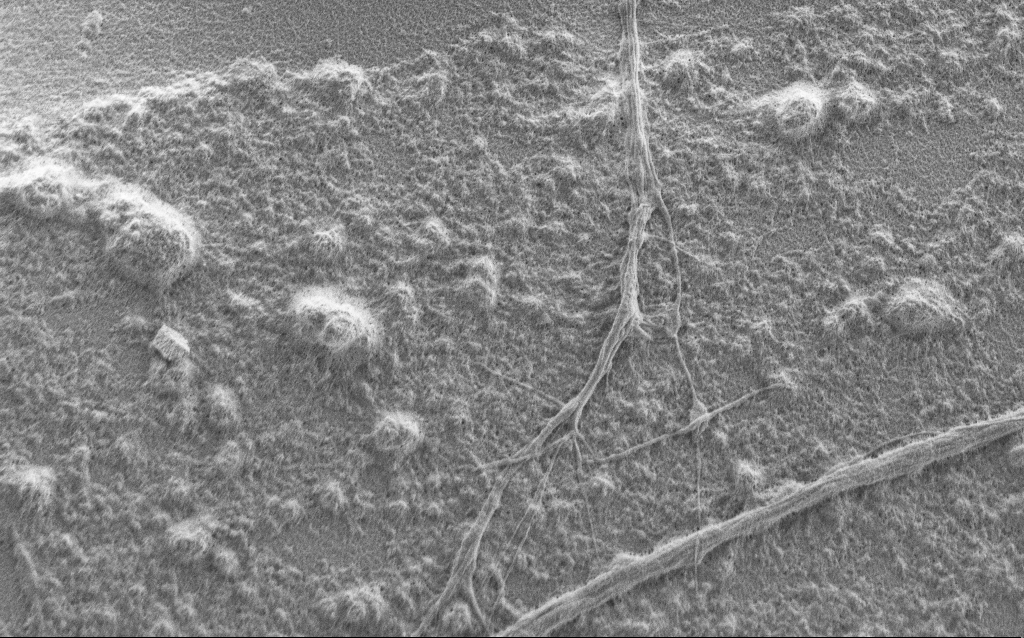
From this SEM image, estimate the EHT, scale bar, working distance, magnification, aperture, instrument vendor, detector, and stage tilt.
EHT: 1 kV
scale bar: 2000 nm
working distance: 6 mm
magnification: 7.5 K X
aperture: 30 µm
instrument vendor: Zeiss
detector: SE2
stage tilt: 0°